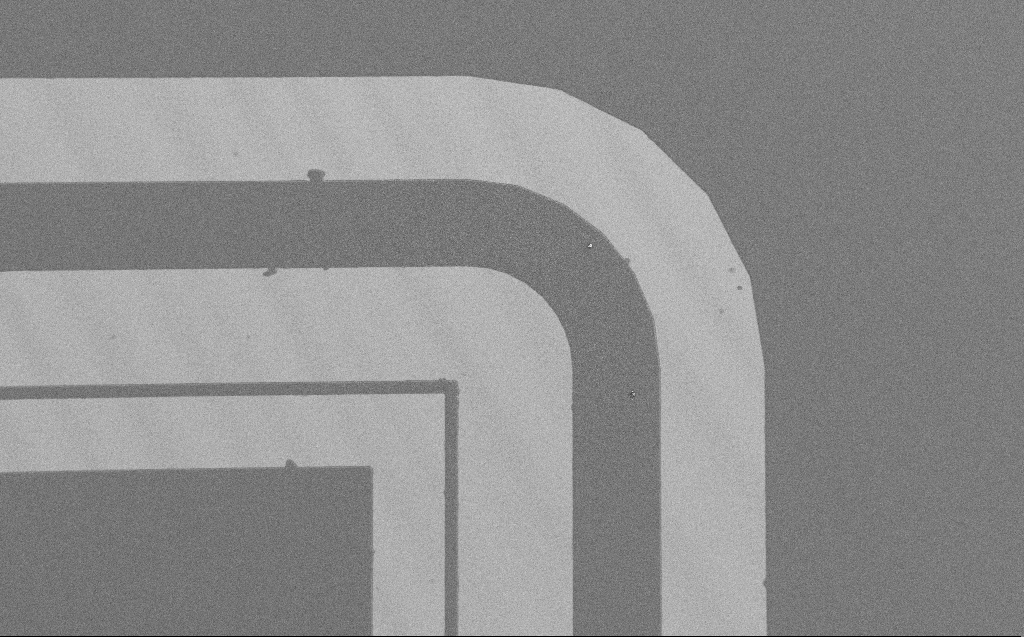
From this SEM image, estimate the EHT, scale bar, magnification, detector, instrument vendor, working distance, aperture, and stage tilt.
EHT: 1.2 kV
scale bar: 100000 nm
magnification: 0.391 K X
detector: SE2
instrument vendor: Zeiss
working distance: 4 mm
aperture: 30 µm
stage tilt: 0°